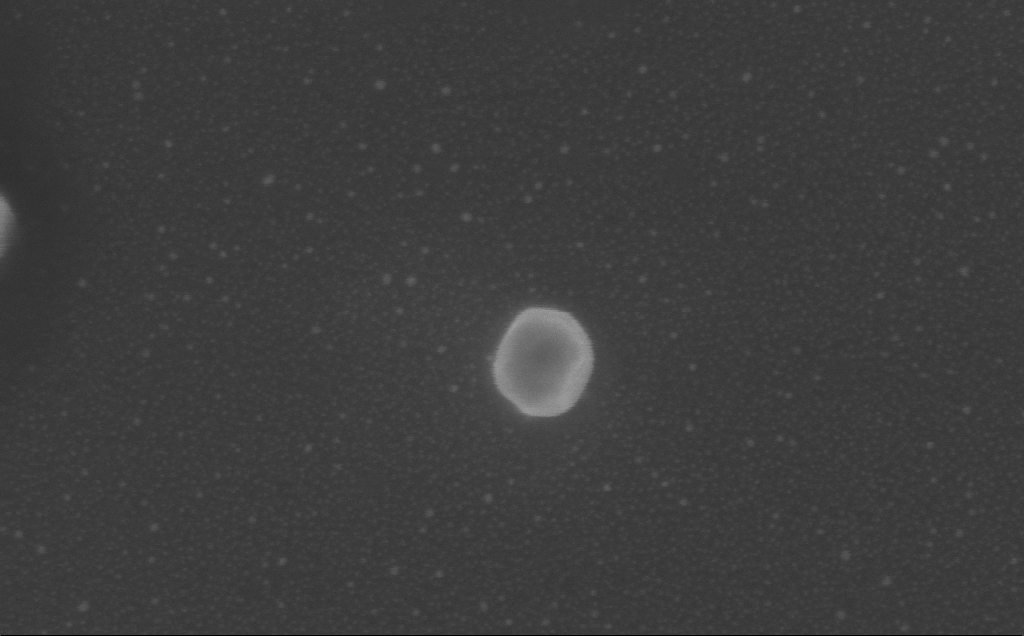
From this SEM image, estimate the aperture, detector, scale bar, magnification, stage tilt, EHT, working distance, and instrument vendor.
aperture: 30 µm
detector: InLens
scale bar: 200 nm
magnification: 243.03 K X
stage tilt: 0°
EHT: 5 kV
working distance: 3 mm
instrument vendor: Zeiss